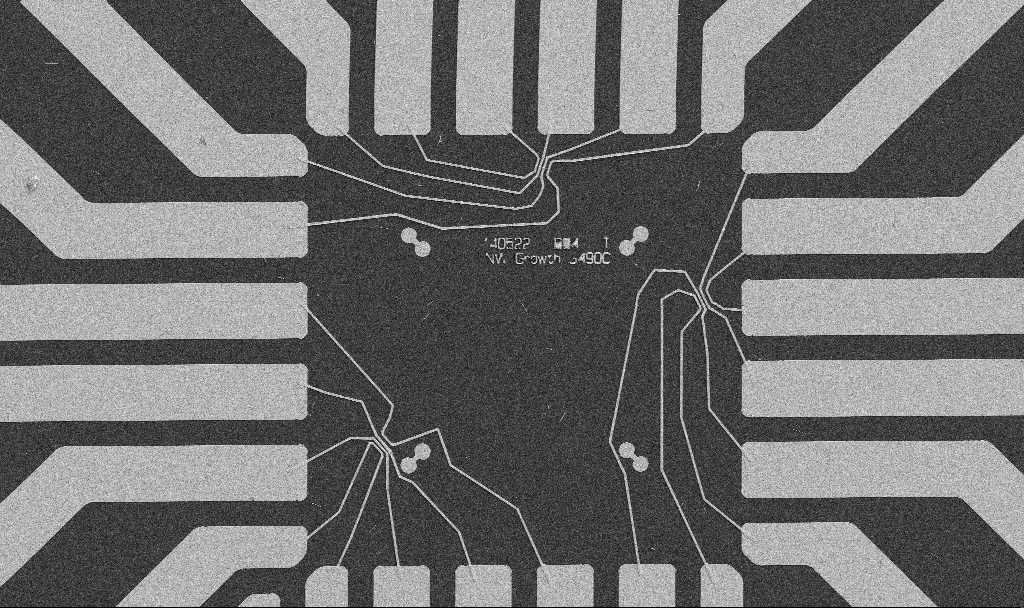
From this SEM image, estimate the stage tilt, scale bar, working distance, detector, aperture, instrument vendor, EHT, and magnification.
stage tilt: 0°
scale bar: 20000 nm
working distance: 10.7 mm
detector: SE2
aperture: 30 µm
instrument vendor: Zeiss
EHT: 5 kV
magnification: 1 K X